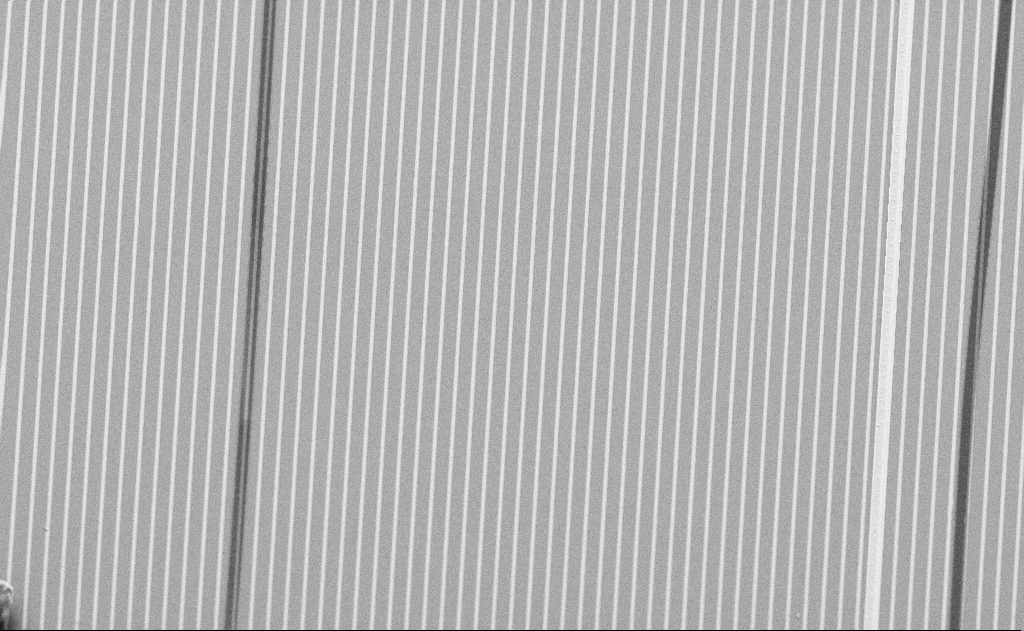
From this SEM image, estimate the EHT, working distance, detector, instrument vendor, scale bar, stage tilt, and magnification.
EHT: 5 kV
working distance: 11 mm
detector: SE2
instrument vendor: Zeiss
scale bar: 20000 nm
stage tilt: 50°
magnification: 1.58 K X